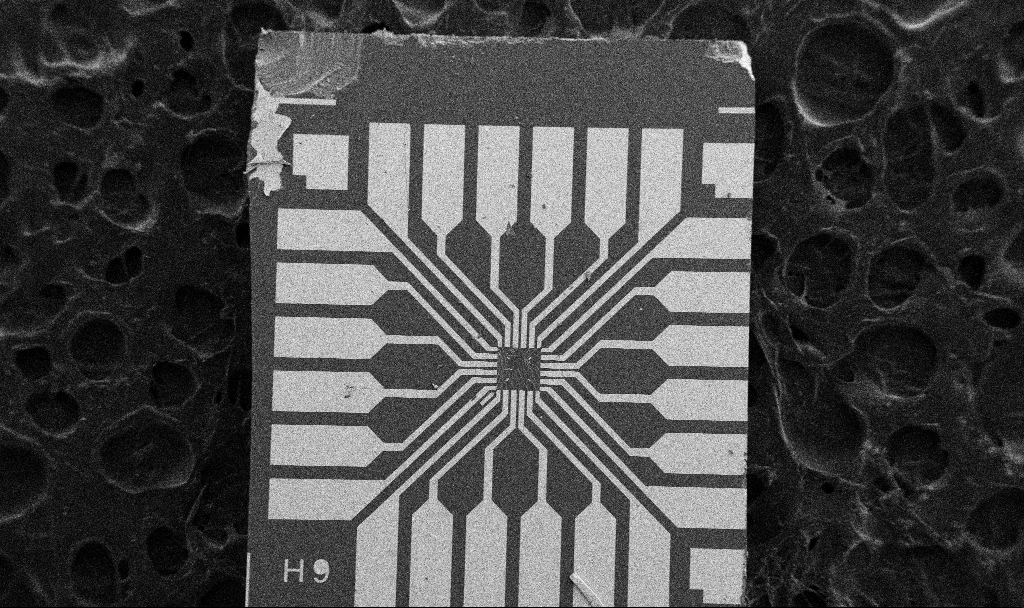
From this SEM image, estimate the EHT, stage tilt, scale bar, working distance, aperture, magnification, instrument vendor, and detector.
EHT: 5 kV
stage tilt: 0°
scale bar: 200000 nm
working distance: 10.6 mm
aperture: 30 µm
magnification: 0.1 K X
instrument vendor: Zeiss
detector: SE2